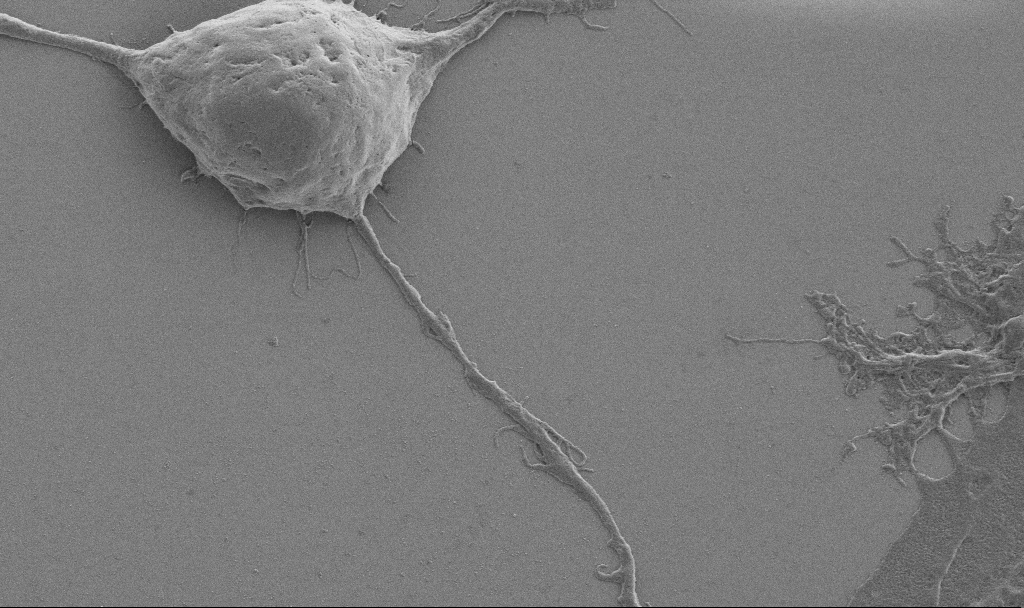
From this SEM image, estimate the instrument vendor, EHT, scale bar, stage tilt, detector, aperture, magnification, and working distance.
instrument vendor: Zeiss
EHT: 0.9 kV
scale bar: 2000 nm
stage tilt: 0°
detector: SE2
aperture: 30 µm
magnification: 10 K X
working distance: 6.9 mm